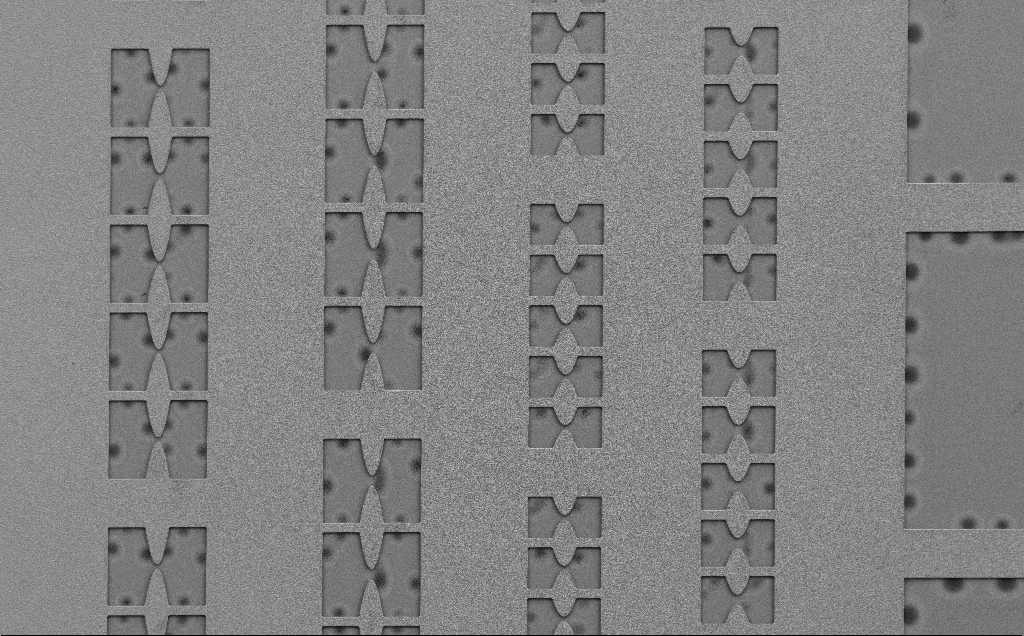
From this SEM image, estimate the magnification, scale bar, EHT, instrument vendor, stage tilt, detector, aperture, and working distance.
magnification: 0.363 K X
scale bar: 100000 nm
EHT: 5 kV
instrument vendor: Zeiss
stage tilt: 0°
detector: SE2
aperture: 30 µm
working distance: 6 mm